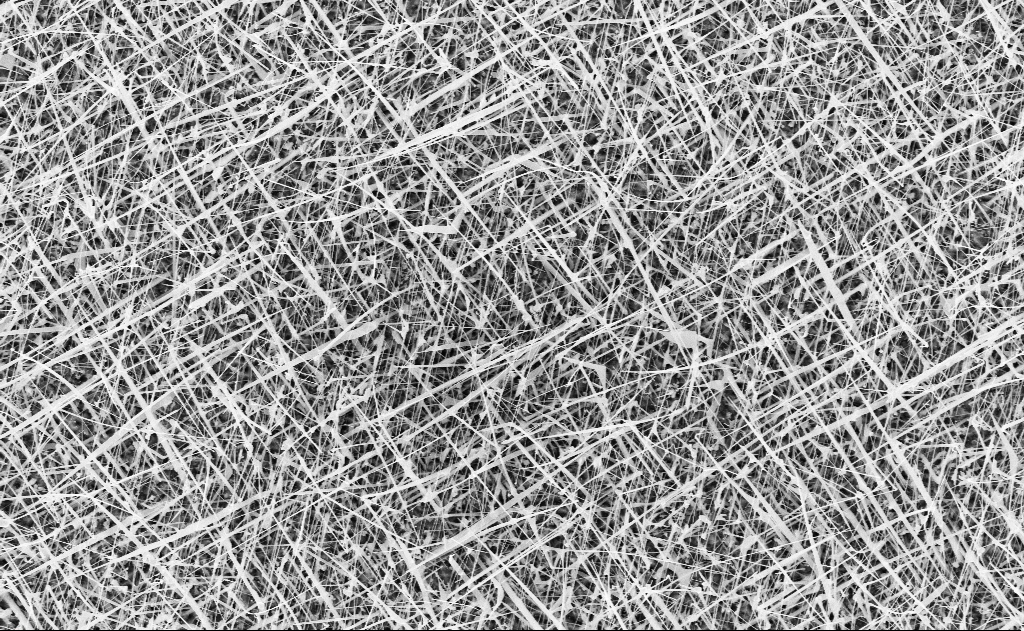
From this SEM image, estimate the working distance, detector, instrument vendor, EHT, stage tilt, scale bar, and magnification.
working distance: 20 mm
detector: InLens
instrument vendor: Zeiss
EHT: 10 kV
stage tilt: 0°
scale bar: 2000 nm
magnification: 10 K X